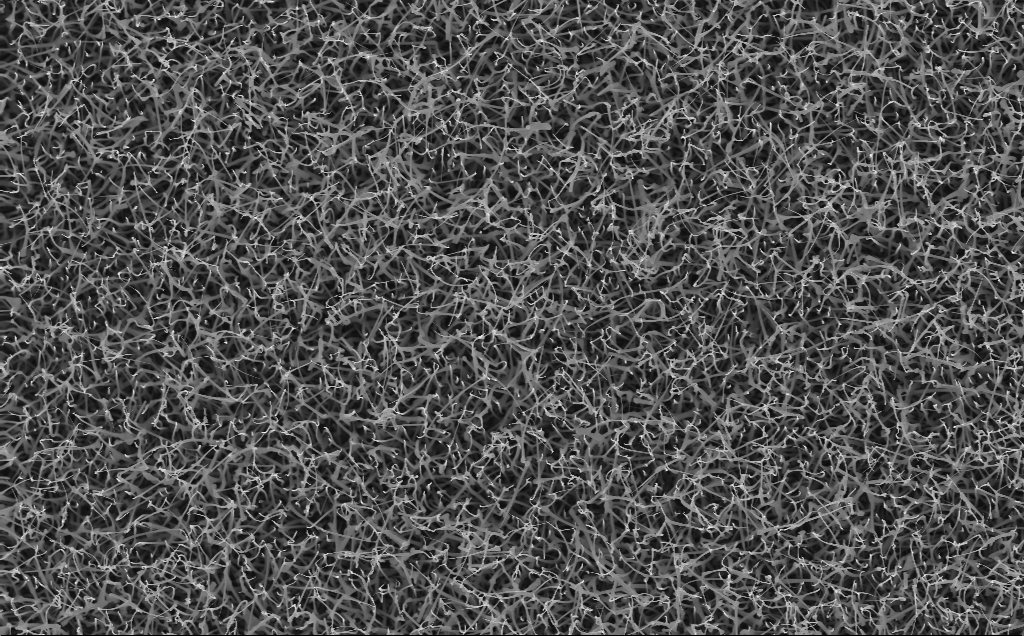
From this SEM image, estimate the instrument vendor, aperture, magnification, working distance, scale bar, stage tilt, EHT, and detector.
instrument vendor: Zeiss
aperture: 30 µm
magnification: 10 K X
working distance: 5 mm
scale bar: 2000 nm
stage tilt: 0°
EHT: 10 kV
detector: InLens